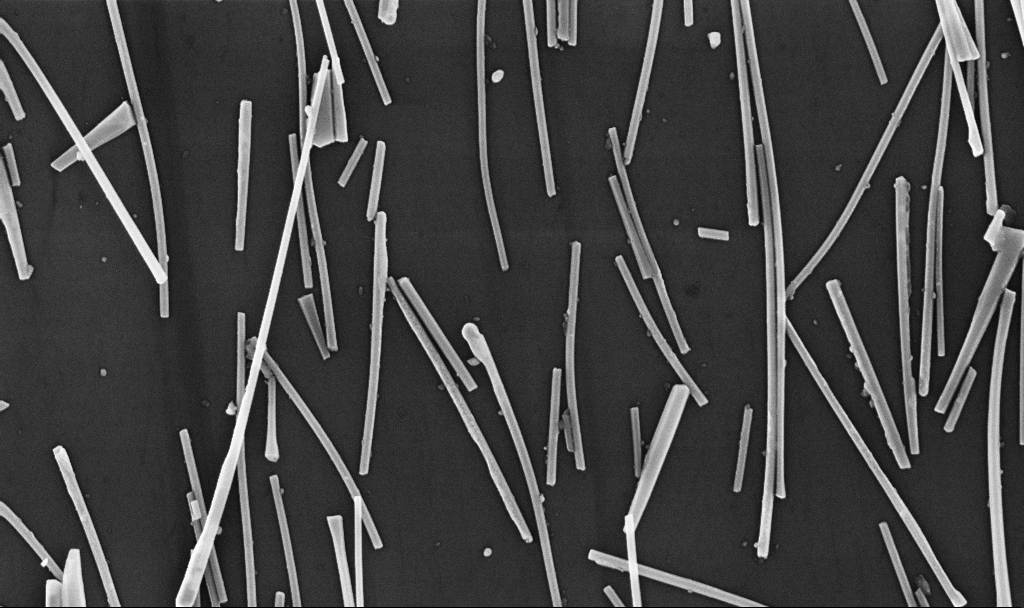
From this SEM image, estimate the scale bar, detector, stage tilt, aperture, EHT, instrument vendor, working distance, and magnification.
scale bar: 1000 nm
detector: InLens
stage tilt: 0°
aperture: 30 µm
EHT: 10 kV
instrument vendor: Zeiss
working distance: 6.7 mm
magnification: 29.32 K X